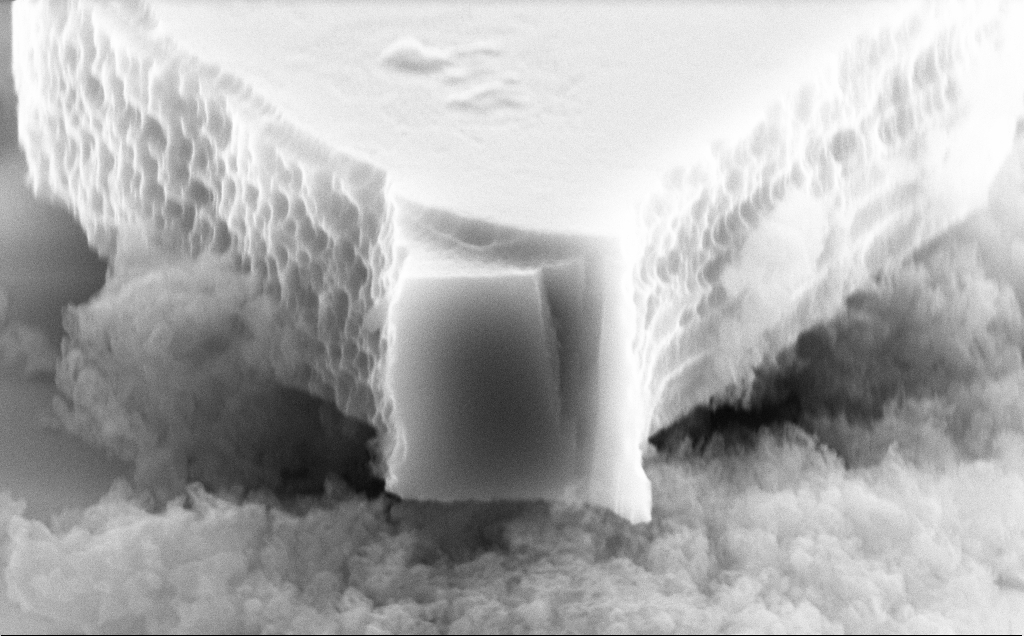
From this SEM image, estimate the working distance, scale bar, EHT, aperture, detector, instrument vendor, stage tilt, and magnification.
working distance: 9 mm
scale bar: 1000 nm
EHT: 10 kV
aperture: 30 µm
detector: SE2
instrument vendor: Zeiss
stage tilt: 70°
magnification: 39.29 K X